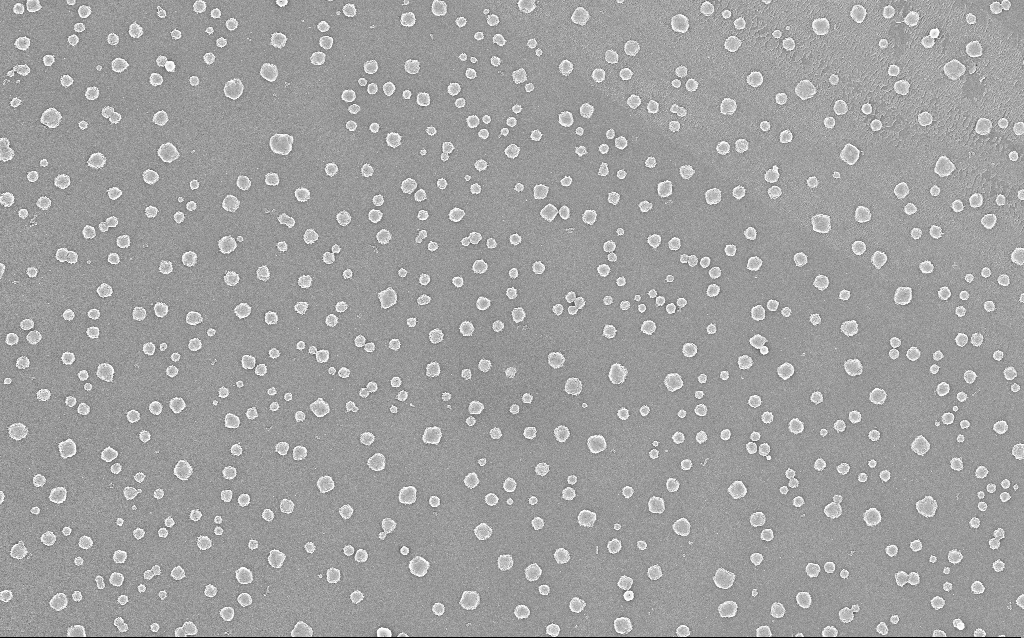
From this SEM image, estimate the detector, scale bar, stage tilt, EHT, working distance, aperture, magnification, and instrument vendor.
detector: InLens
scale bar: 2000 nm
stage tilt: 0°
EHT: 20 kV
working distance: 1.8 mm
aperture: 30 µm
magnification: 10 K X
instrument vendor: Zeiss